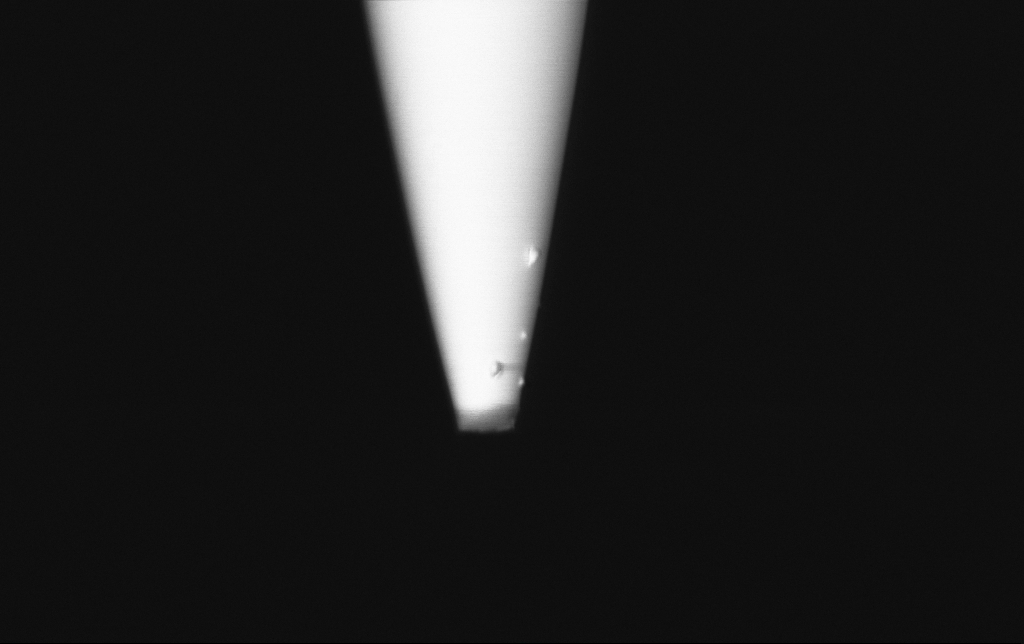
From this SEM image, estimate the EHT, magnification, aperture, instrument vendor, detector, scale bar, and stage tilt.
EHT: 1 kV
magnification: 25 K X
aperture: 30 µm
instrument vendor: Zeiss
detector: InLens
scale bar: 1000 nm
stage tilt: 0°